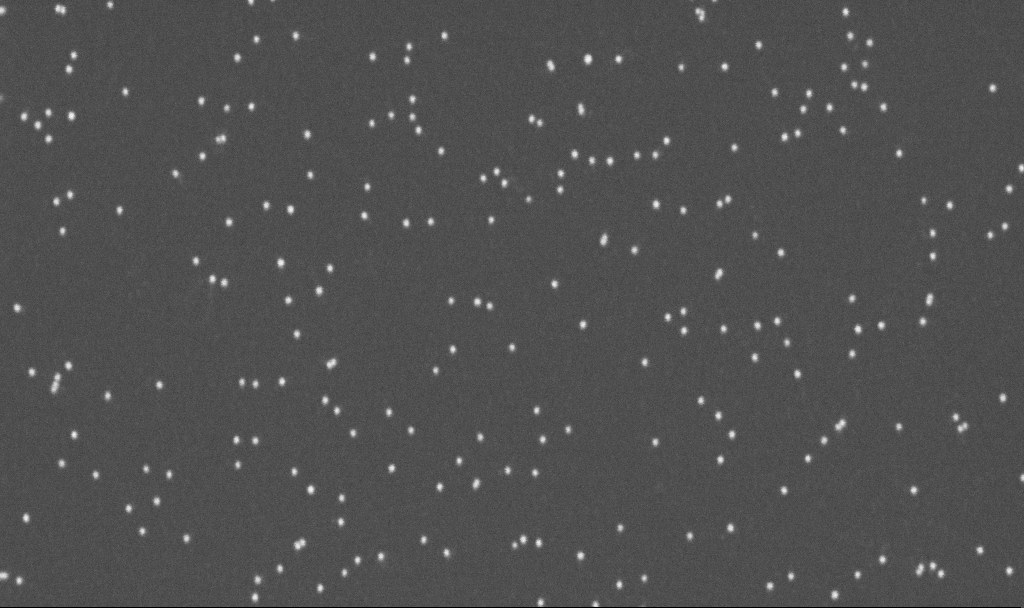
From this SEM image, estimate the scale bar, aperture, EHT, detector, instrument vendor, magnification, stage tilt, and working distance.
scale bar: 200 nm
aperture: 30 µm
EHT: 10 kV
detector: InLens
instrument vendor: Zeiss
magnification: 200 K X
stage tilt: -0°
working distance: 3.3 mm